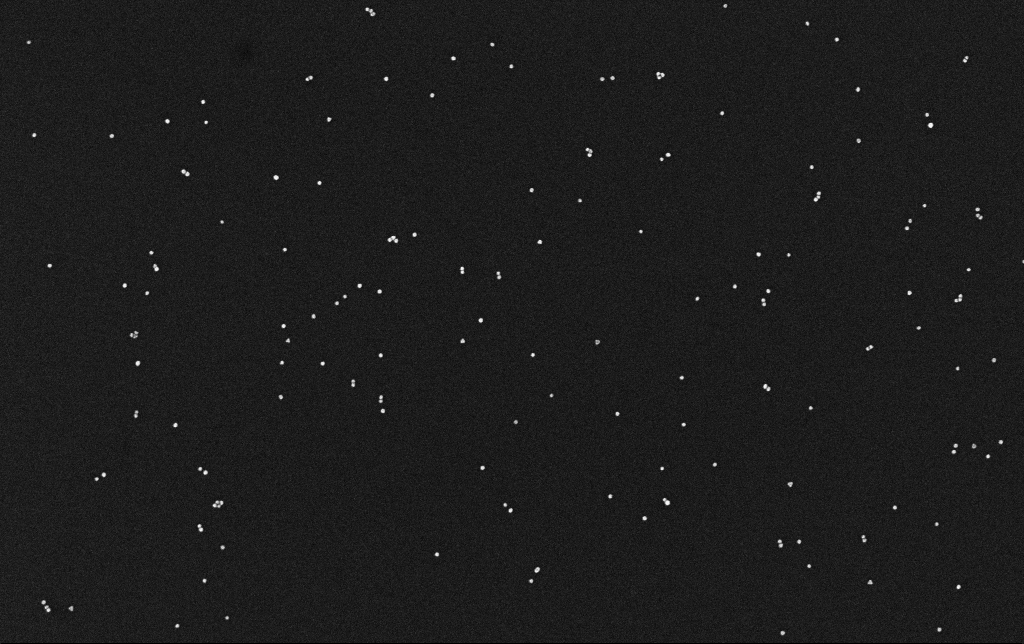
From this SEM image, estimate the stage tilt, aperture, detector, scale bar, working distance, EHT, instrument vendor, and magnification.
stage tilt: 0°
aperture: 30 µm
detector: InLens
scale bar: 200 nm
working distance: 3.1 mm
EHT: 10 kV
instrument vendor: Zeiss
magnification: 100 K X